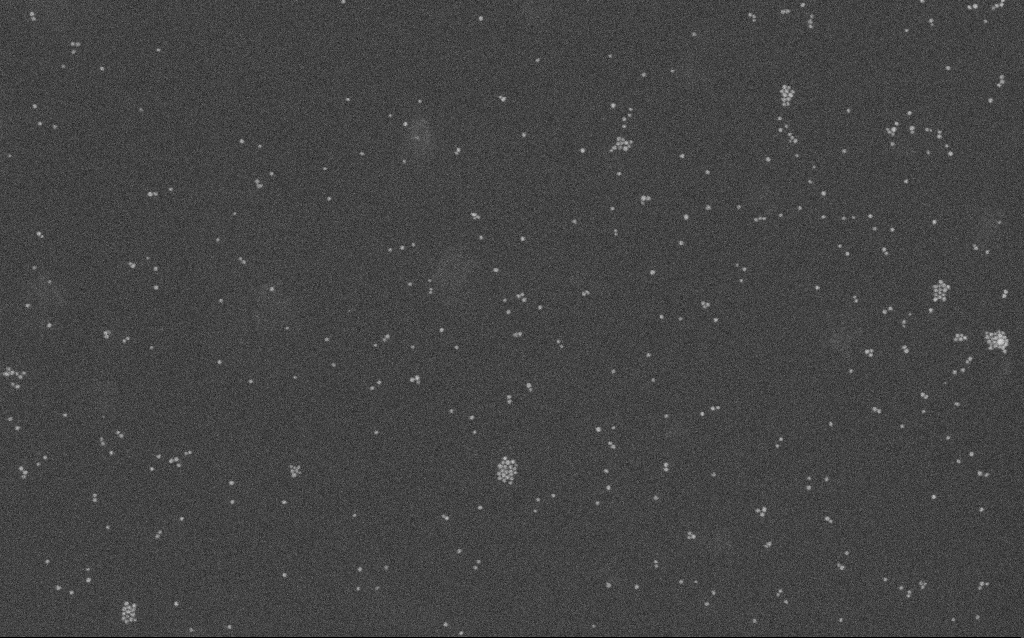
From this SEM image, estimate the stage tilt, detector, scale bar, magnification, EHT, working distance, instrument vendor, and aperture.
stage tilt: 0°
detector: SE2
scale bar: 200 nm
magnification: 100 K X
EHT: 10 kV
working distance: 2.1 mm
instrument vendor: Zeiss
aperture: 30 µm